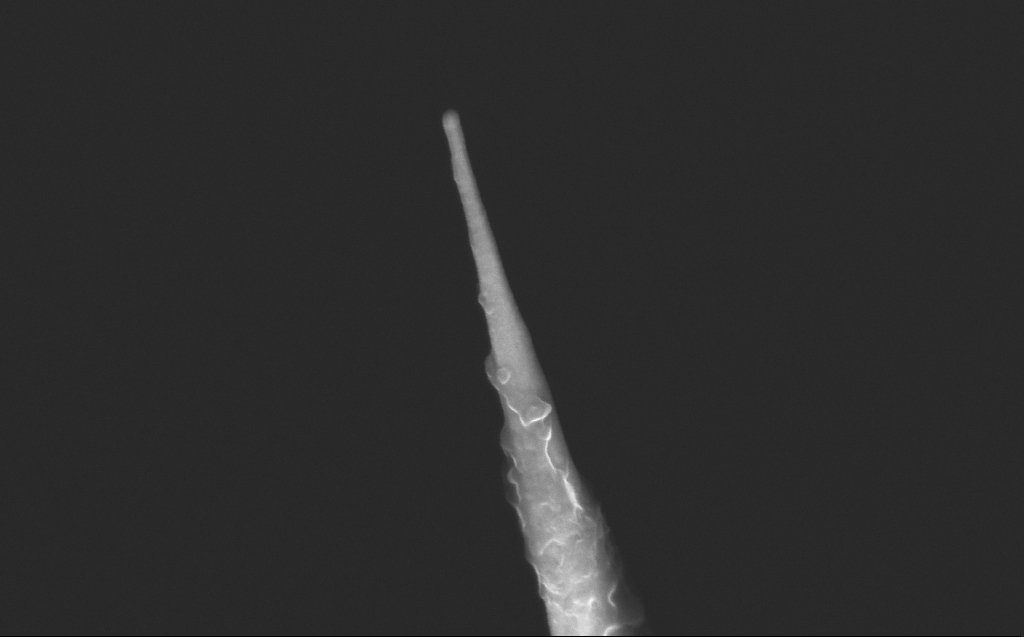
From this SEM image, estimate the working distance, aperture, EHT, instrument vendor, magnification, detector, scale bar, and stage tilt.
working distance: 6 mm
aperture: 30 µm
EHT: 10 kV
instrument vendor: Zeiss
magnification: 106.94 K X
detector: InLens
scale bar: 200 nm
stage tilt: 40°